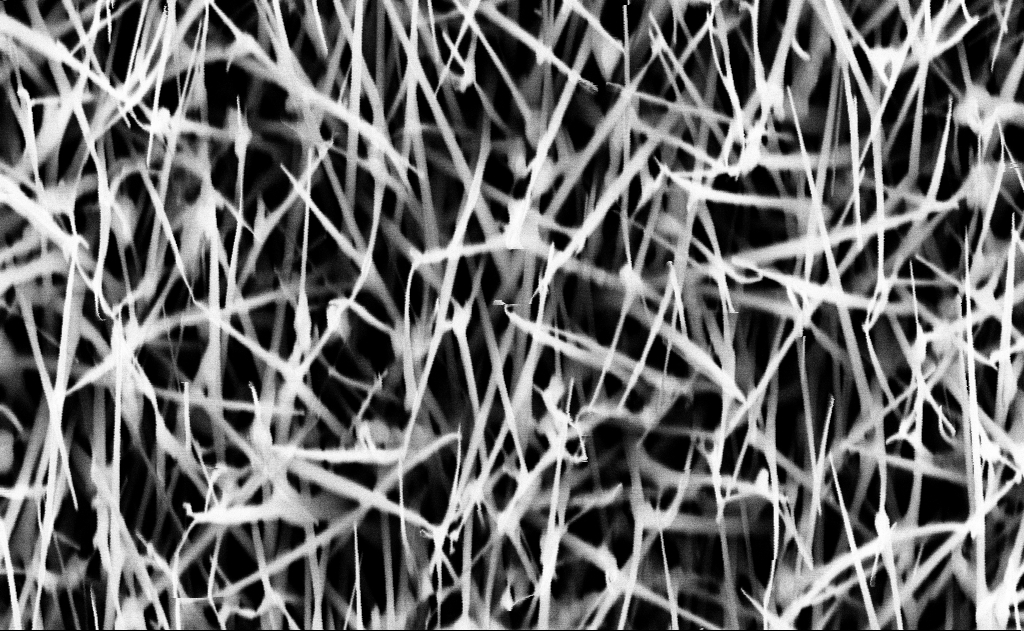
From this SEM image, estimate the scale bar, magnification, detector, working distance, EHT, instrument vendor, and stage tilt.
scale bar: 1000 nm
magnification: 40 K X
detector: InLens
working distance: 16 mm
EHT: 10 kV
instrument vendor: Zeiss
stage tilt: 0°